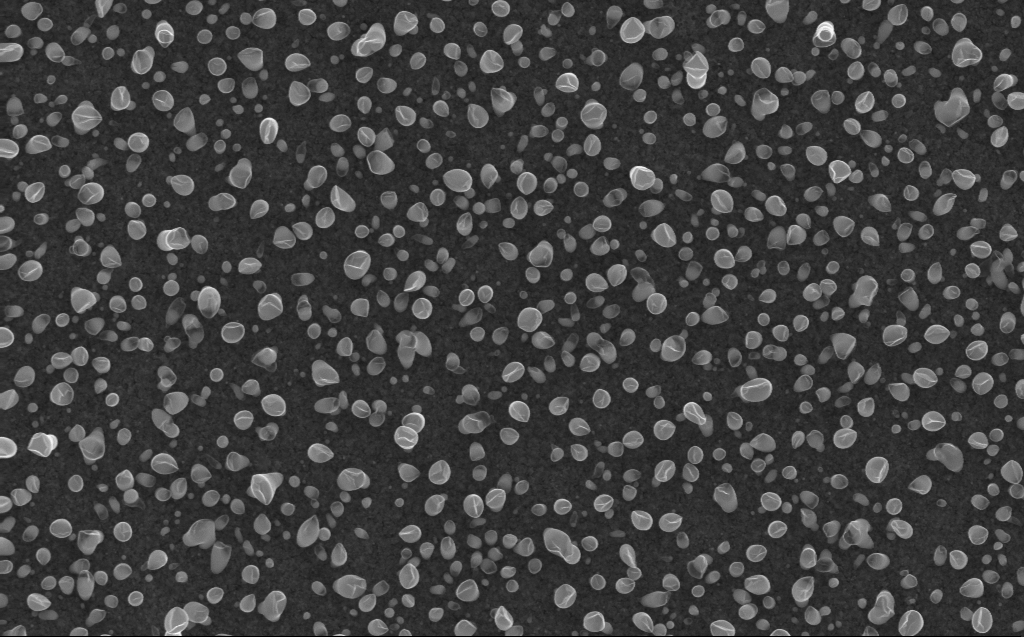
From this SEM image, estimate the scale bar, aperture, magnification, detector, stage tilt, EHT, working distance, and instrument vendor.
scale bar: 1000 nm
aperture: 30 µm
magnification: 50 K X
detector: InLens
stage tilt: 0°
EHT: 10 kV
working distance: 3 mm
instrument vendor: Zeiss